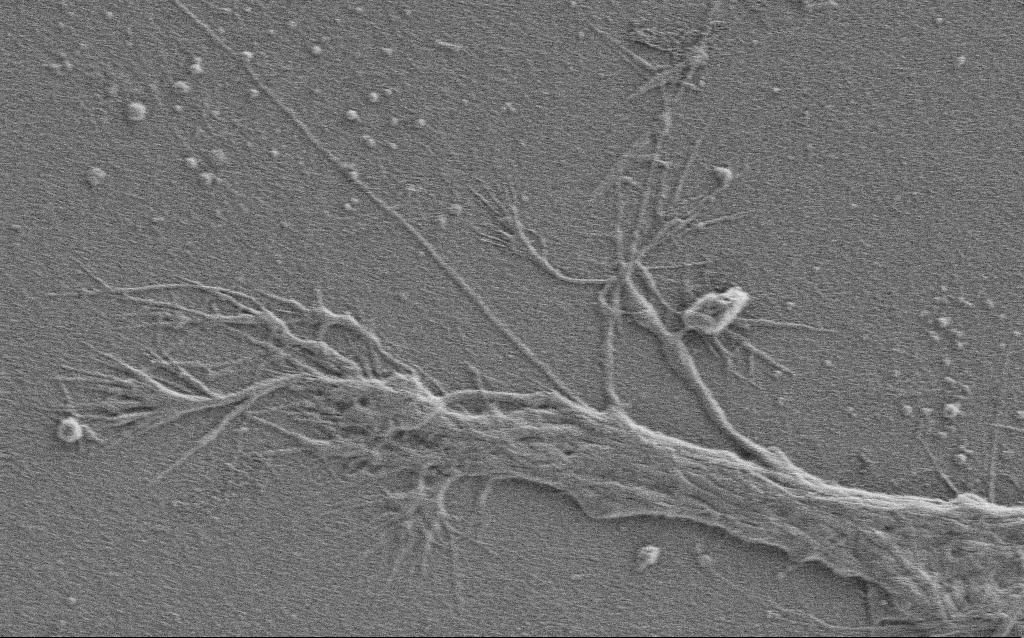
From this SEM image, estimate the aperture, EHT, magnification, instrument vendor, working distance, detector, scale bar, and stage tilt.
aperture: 30 µm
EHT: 0.9 kV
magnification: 5 K X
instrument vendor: Zeiss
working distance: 3.6 mm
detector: SE2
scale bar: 10000 nm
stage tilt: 0°